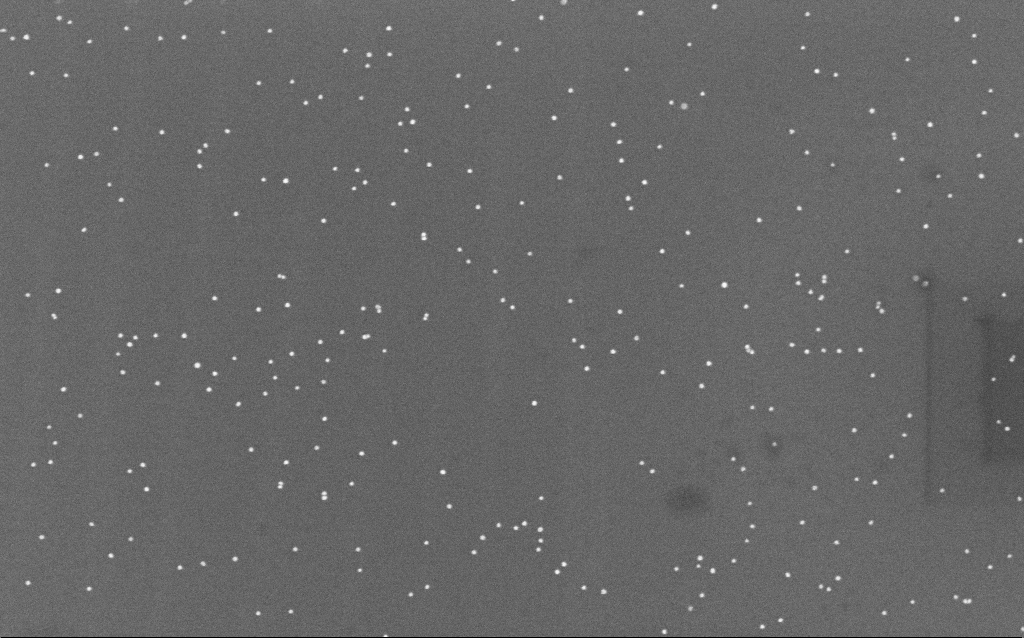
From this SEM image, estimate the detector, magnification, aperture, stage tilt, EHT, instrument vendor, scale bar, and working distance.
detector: InLens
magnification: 100 K X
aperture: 30 µm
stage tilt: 0°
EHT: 10 kV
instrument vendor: Zeiss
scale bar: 200 nm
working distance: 6.6 mm